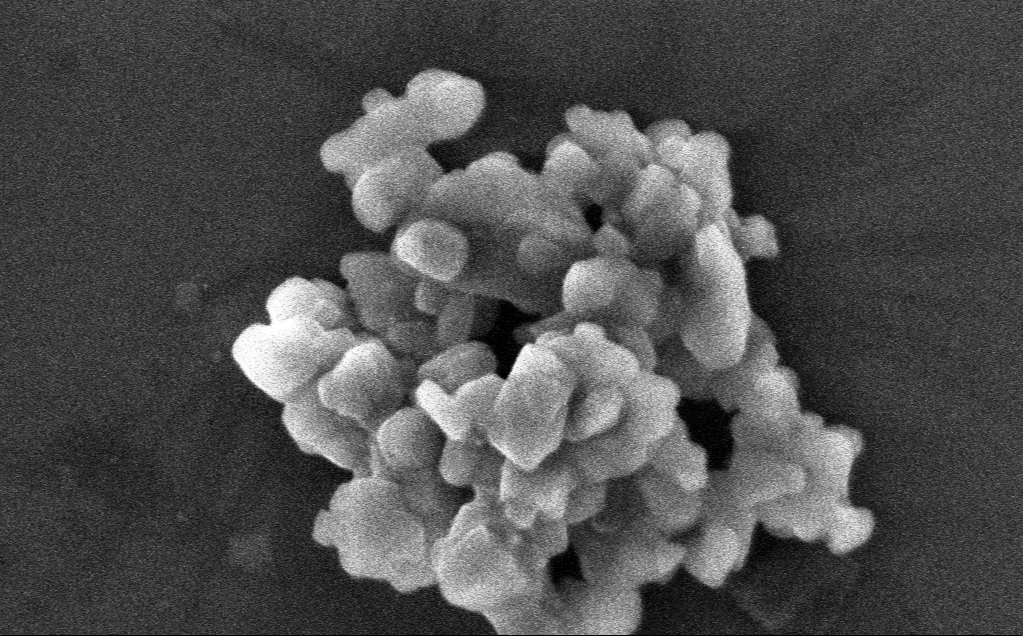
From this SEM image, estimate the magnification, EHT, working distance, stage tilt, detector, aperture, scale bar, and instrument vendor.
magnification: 189.31 K X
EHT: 3 kV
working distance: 3 mm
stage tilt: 0°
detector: InLens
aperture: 30 µm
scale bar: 200 nm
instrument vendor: Zeiss